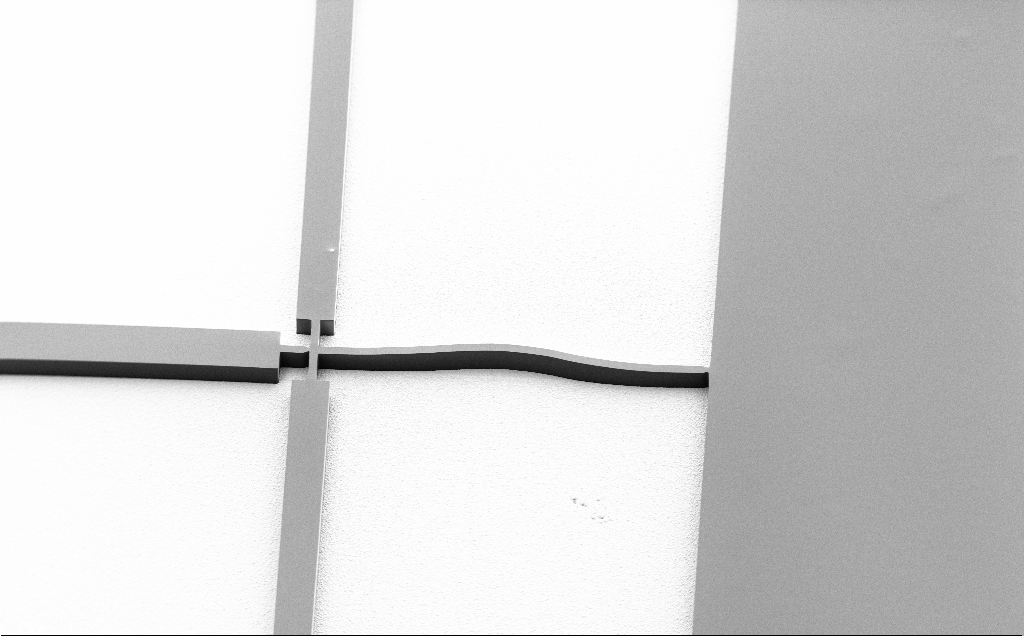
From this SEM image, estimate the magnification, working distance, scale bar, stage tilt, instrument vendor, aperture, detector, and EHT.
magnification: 0.281 K X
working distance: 8 mm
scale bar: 200000 nm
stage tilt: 45°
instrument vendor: Zeiss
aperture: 30 µm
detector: SE2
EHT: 1.5 kV